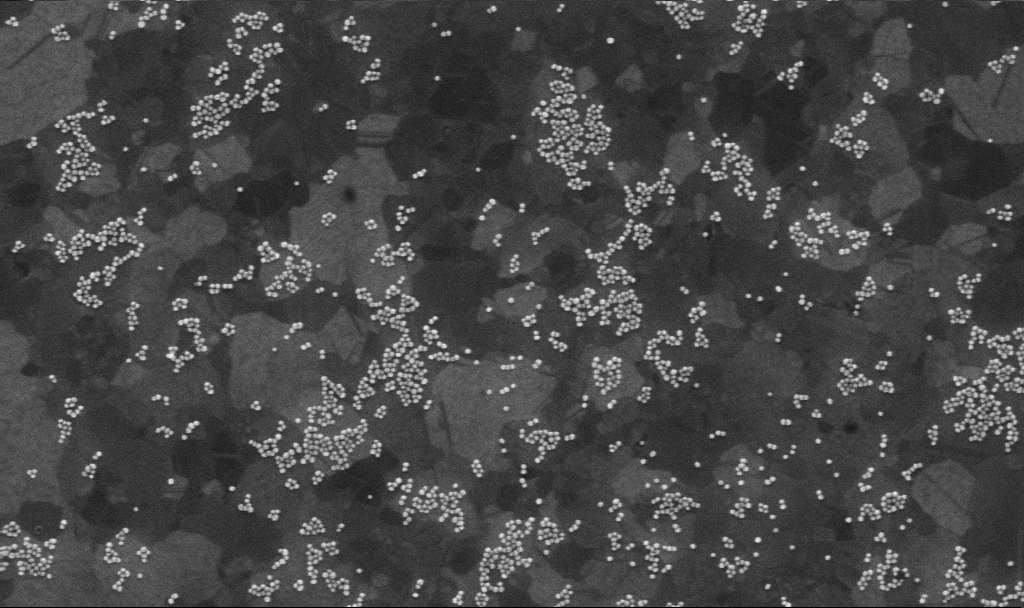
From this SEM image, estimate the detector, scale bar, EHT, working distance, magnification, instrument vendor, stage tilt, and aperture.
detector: InLens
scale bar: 200 nm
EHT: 10 kV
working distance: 3.8 mm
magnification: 103.66 K X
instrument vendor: Zeiss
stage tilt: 0°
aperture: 30 µm